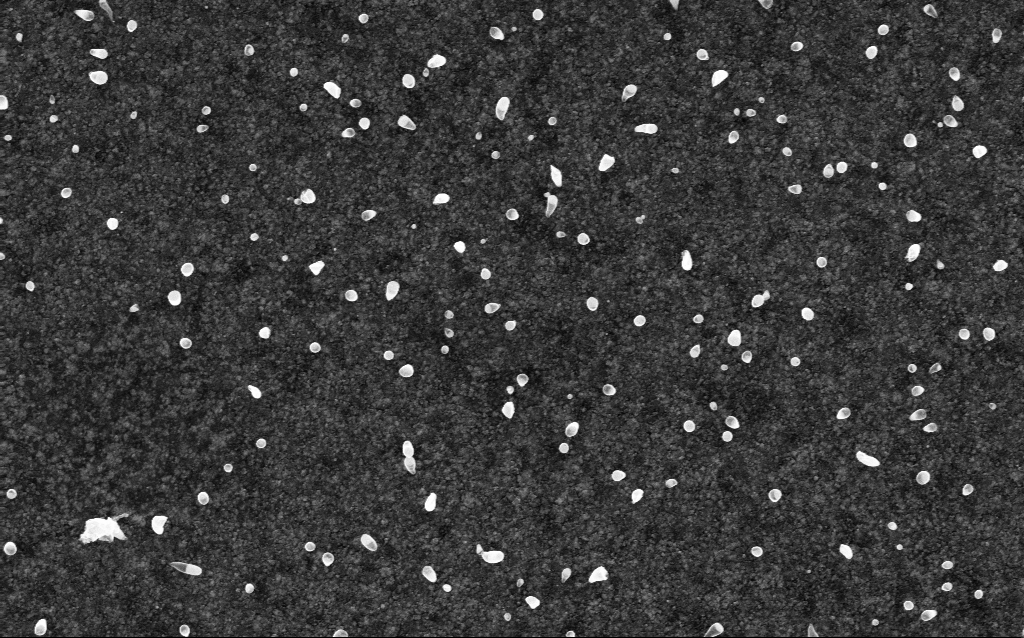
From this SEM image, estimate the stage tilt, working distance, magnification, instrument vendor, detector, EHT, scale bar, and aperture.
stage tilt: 0°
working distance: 2.1 mm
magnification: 50 K X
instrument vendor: Zeiss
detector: InLens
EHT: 5 kV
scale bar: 1000 nm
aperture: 30 µm